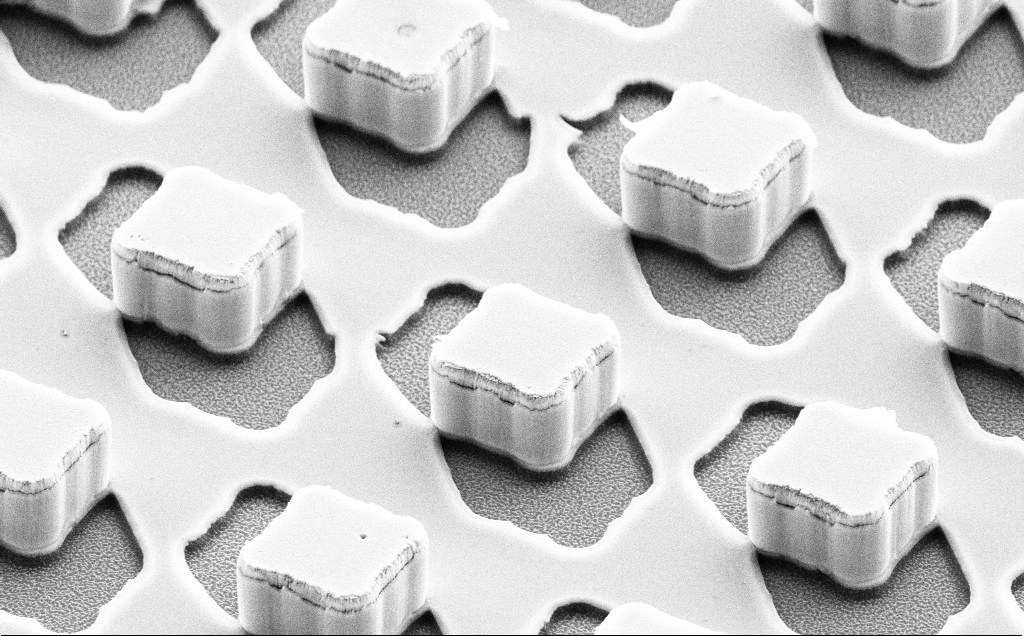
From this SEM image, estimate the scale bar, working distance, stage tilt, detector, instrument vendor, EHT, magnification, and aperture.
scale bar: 10000 nm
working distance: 11 mm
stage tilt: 50°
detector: SE2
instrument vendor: Zeiss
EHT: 10 kV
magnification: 6.81 K X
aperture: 30 µm